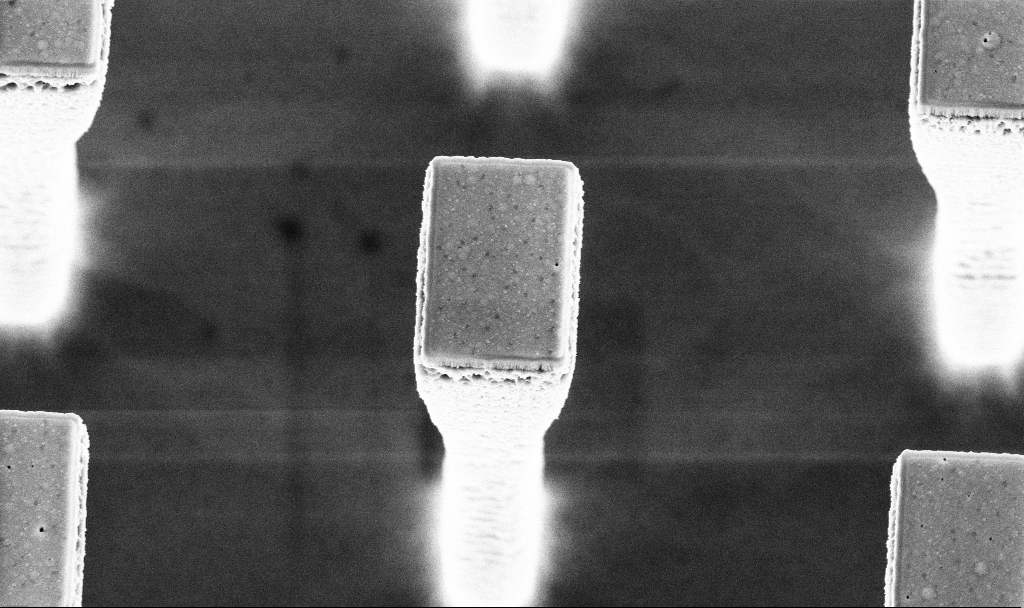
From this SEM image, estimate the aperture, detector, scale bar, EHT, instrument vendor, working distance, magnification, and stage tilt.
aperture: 30 µm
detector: InLens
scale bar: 1000 nm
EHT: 5 kV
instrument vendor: Zeiss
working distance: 4.2 mm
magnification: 17.62 K X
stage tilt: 20.2°